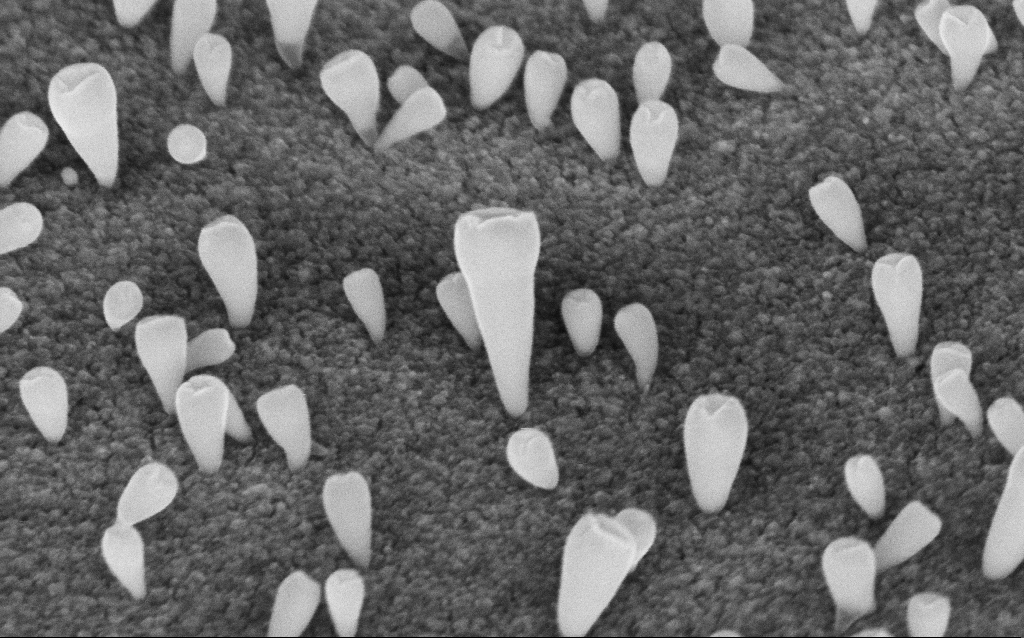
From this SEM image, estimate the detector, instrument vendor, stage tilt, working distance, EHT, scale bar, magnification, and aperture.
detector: InLens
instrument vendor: Zeiss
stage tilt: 42.7°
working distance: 6 mm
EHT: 5 kV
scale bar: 200 nm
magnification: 171.51 K X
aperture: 30 µm